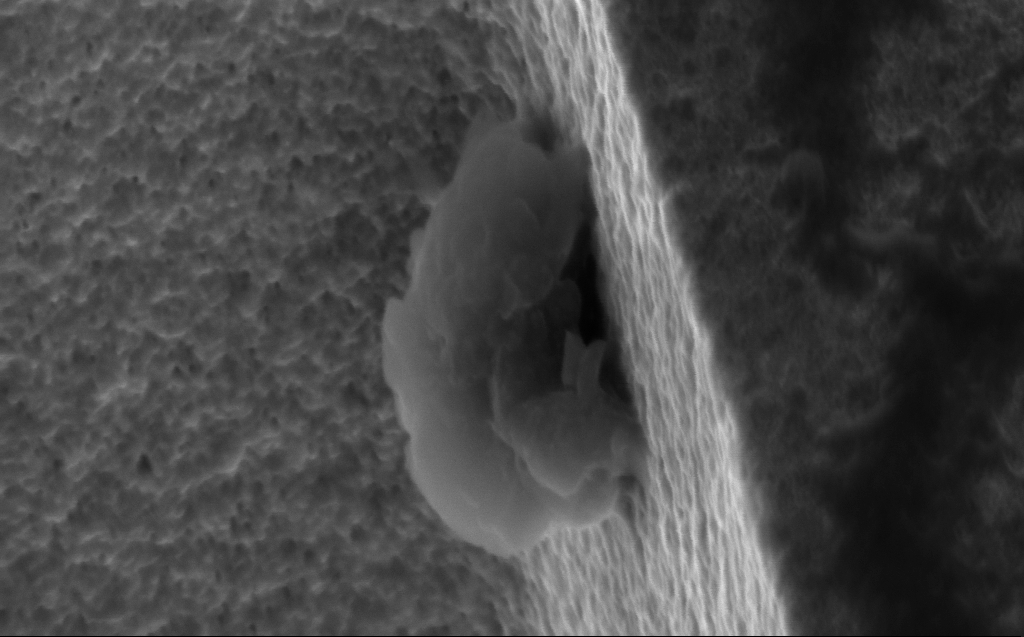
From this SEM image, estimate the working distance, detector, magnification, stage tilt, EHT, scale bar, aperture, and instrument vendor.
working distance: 5 mm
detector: InLens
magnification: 130.59 K X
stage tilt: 45°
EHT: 5 kV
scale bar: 200 nm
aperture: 30 µm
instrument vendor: Zeiss